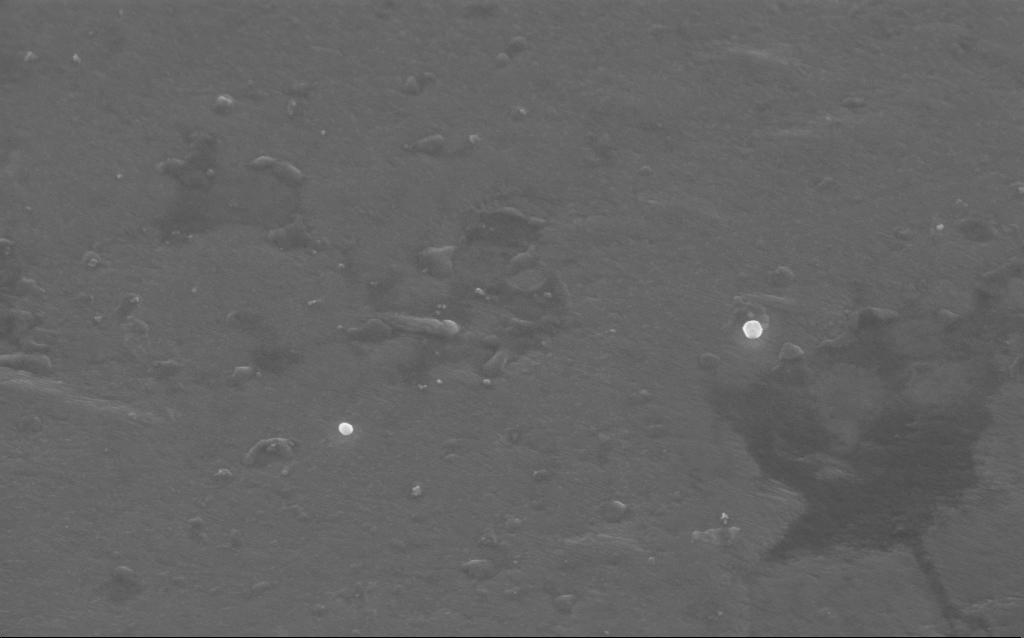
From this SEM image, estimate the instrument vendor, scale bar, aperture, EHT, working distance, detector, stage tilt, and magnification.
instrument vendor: Zeiss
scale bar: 200 nm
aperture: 30 µm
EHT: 5 kV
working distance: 4 mm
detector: InLens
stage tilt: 35°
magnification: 85.33 K X